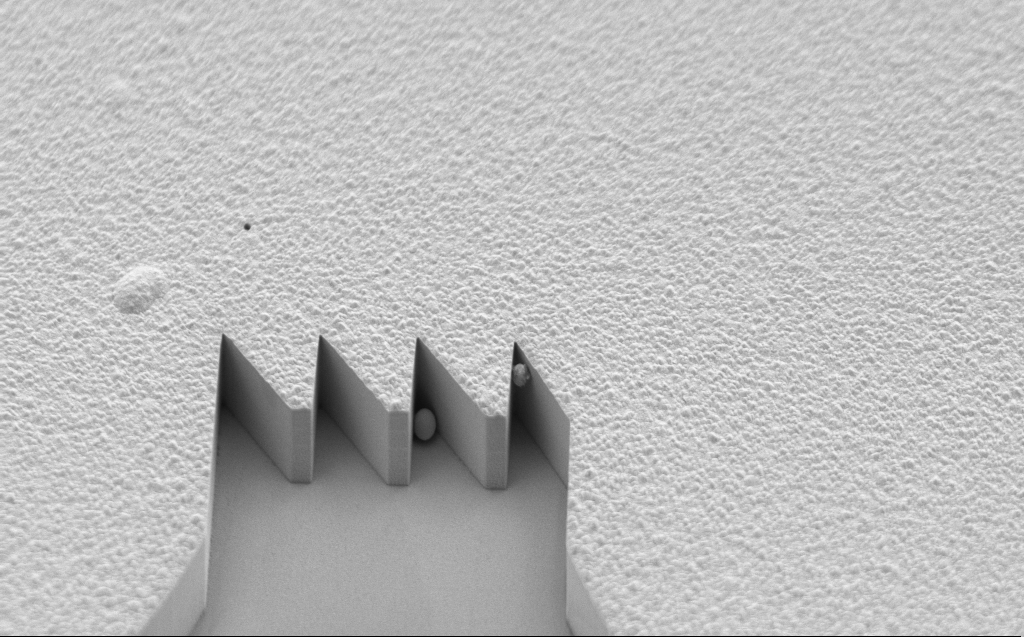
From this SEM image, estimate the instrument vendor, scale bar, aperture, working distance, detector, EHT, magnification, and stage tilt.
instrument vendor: Zeiss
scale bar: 10000 nm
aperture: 120 µm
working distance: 7 mm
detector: SE2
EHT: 10 kV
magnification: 3.14 K X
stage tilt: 45.1°